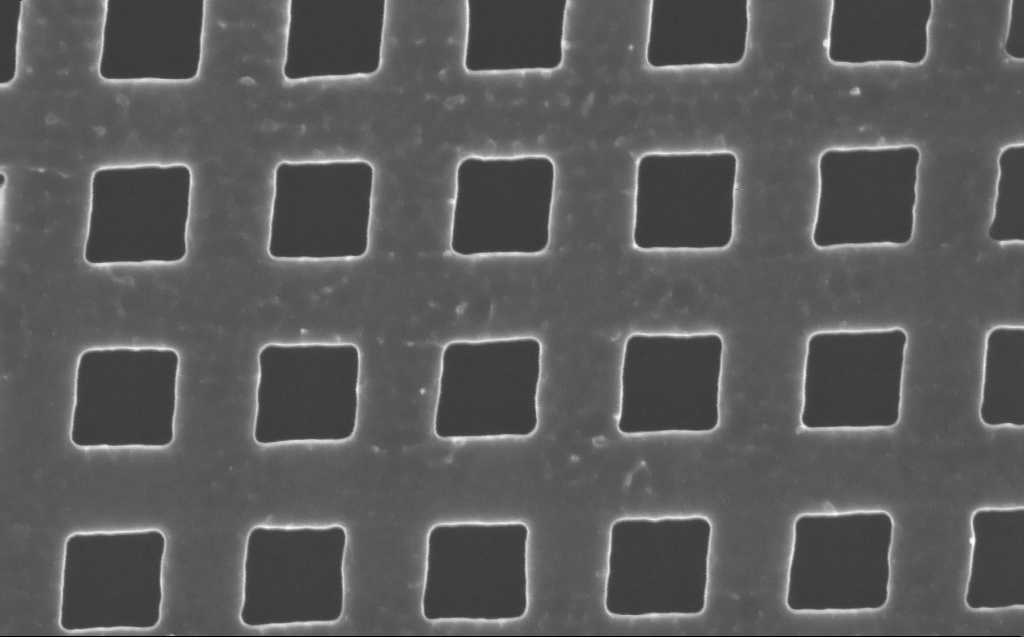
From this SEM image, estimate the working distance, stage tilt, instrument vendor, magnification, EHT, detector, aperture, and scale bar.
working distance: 4 mm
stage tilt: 0°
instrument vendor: Zeiss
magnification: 135 K X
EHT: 10 kV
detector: InLens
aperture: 30 µm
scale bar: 100 nm